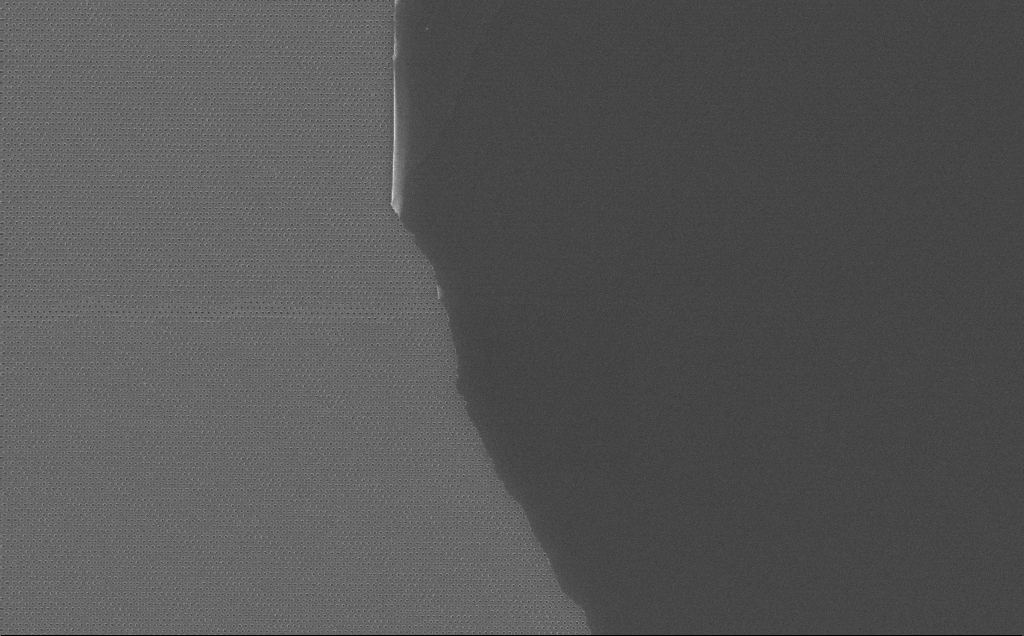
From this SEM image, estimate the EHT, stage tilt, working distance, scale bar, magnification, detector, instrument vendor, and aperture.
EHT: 10 kV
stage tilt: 0°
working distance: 7 mm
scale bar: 2000 nm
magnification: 8.94 K X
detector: InLens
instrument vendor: Zeiss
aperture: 30 µm